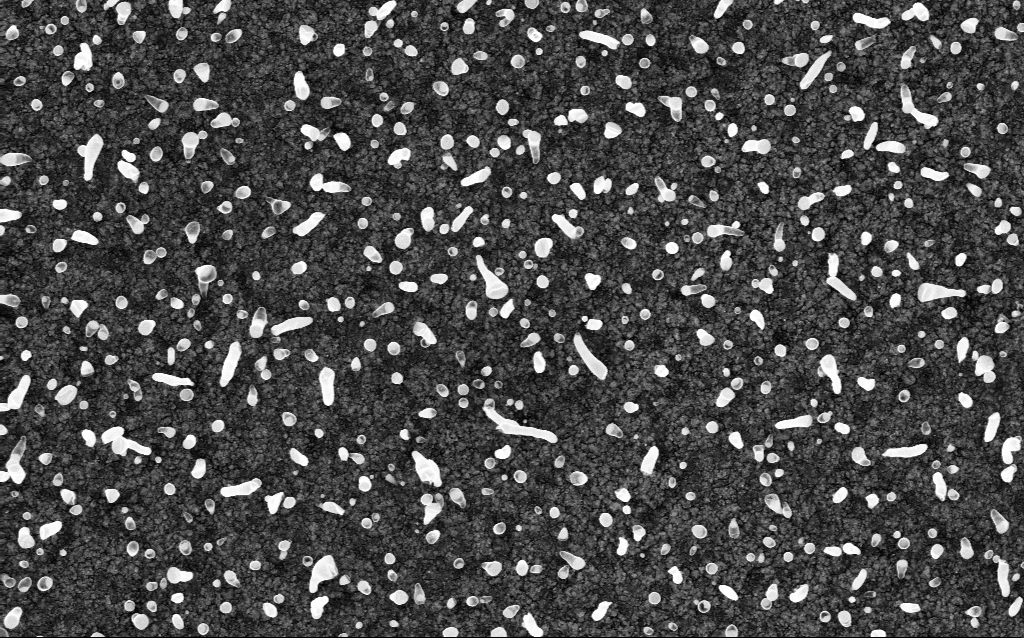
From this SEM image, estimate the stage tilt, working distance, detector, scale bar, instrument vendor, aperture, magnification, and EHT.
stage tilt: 0°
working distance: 2 mm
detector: InLens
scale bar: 1000 nm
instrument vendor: Zeiss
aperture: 30 µm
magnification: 50 K X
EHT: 5 kV